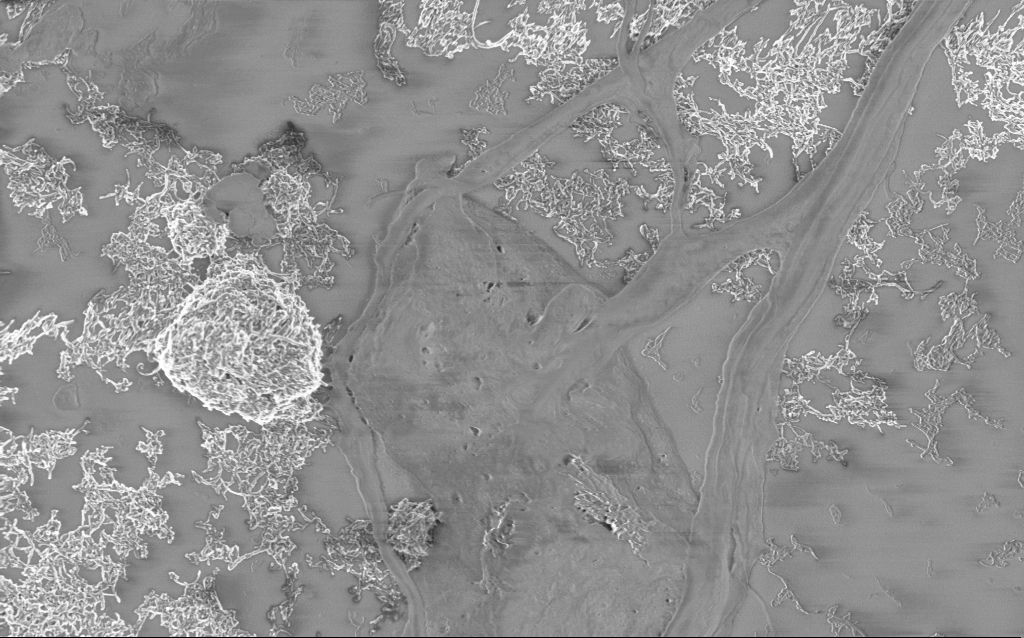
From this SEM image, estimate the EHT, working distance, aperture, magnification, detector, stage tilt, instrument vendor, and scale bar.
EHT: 1 kV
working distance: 4 mm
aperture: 30 µm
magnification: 15 K X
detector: InLens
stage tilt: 0°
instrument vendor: Zeiss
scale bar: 1000 nm